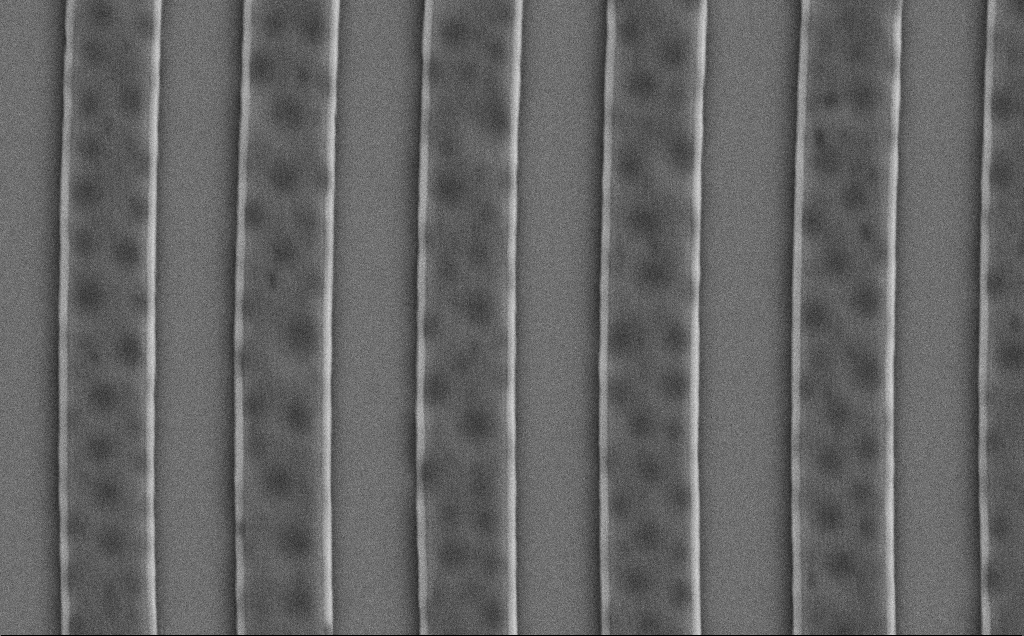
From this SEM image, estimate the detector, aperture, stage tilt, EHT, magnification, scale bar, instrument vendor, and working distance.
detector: SE2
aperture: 30 µm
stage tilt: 0°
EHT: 5 kV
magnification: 25.1 K X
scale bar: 2000 nm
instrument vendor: Zeiss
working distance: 7 mm